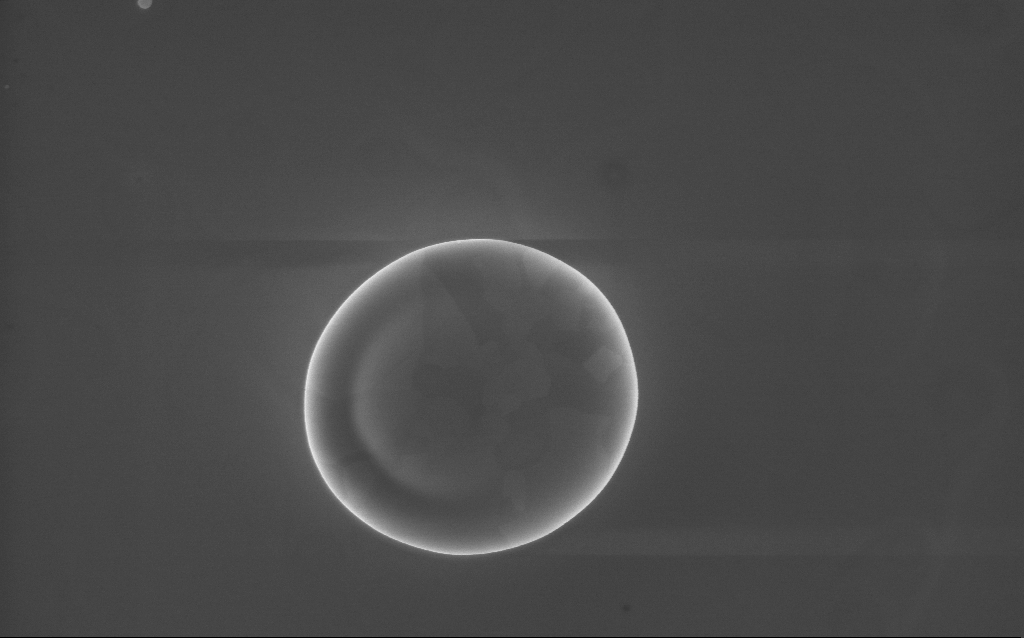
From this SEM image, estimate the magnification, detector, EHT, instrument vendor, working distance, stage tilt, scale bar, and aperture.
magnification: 36 K X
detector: InLens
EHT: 10 kV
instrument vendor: Zeiss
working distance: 3 mm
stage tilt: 0°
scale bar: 1000 nm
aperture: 30 µm